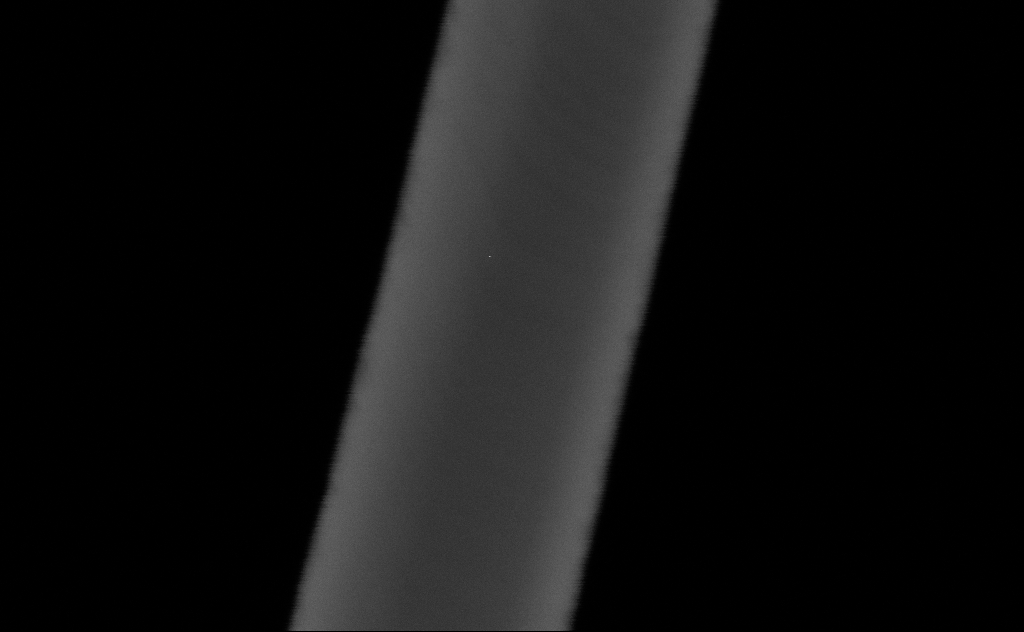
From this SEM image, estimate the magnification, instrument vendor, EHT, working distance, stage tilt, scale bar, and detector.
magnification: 761.93 K X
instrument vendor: Zeiss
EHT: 20 kV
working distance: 8 mm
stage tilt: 0°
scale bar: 20 nm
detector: SE2